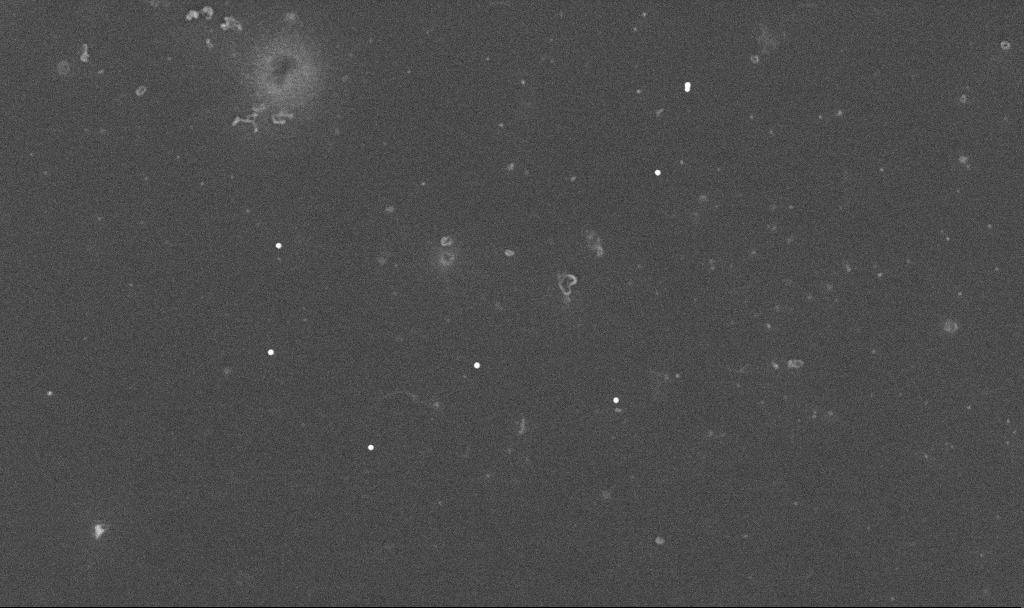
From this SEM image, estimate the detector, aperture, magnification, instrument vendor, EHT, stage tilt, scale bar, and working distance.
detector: InLens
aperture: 30 µm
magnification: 22.42 K X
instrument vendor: Zeiss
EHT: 10 kV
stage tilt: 0°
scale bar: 1000 nm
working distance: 3.4 mm